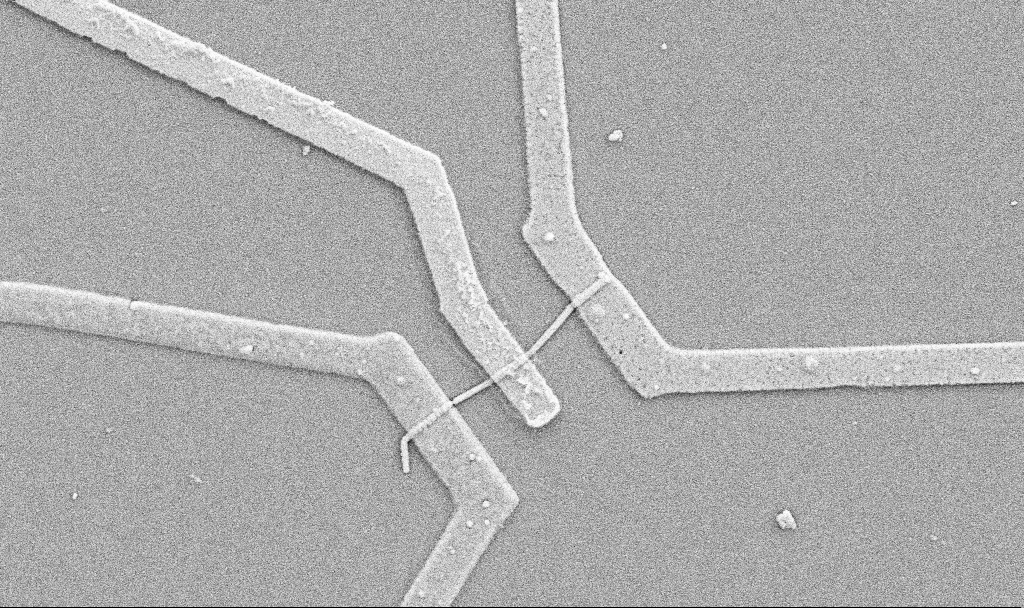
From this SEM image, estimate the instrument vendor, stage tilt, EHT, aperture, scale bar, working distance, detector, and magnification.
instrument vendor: Zeiss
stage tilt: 0°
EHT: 5 kV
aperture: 30 µm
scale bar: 1000 nm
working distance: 10.7 mm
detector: SE2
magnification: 20 K X